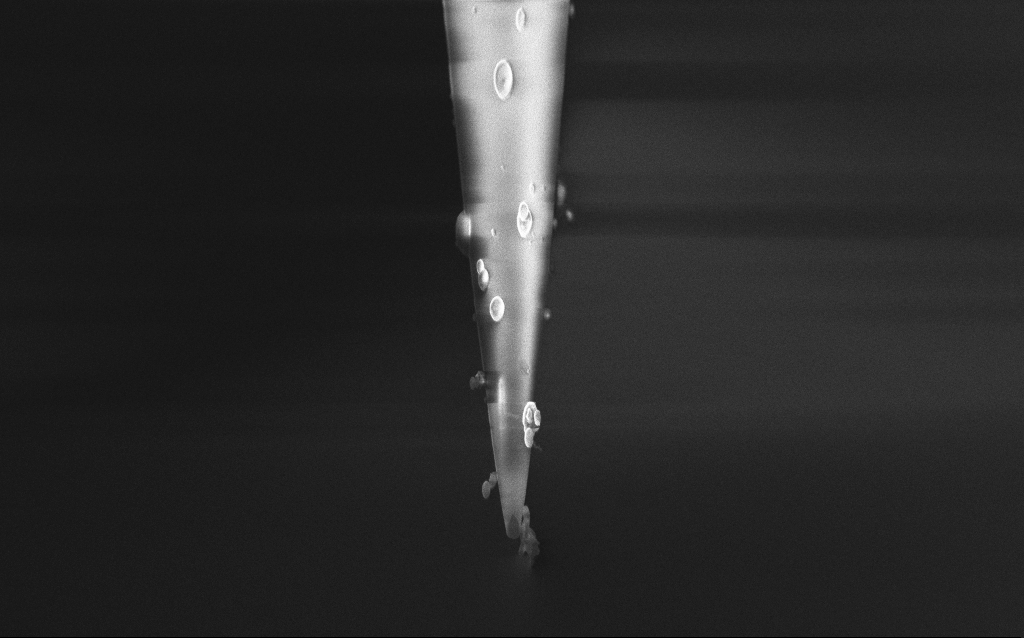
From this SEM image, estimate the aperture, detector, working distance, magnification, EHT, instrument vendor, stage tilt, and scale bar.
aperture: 30 µm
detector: InLens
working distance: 4 mm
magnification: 14.92 K X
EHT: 2 kV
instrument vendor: Zeiss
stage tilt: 45°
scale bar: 1000 nm